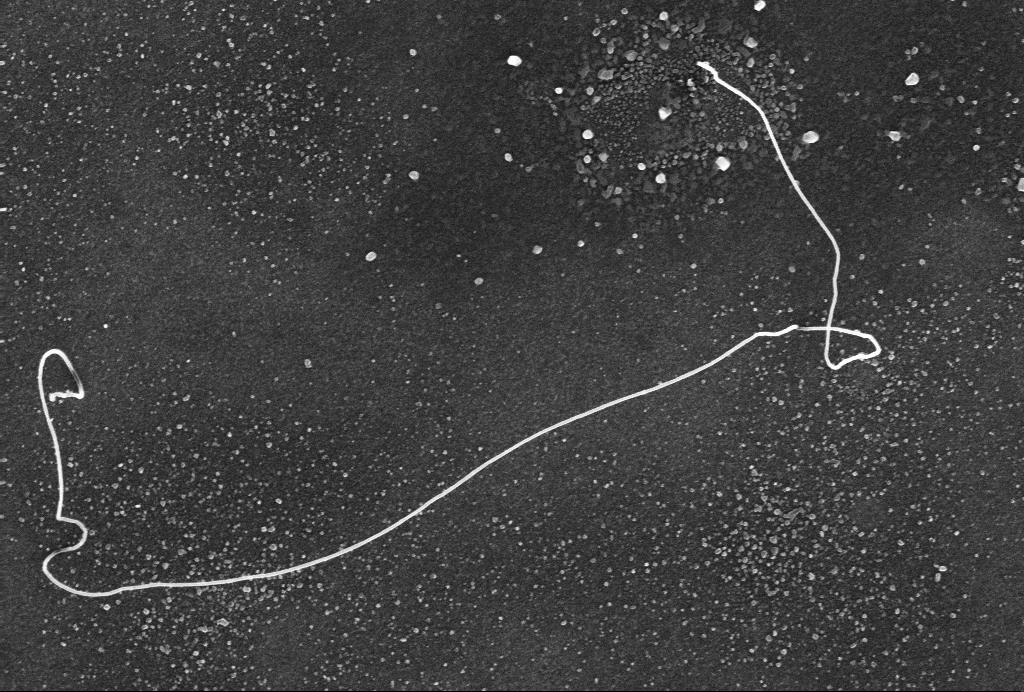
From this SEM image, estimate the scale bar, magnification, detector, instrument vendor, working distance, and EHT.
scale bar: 1000 nm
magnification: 53.44 K X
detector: InLens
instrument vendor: Zeiss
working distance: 3.1 mm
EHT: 10 kV